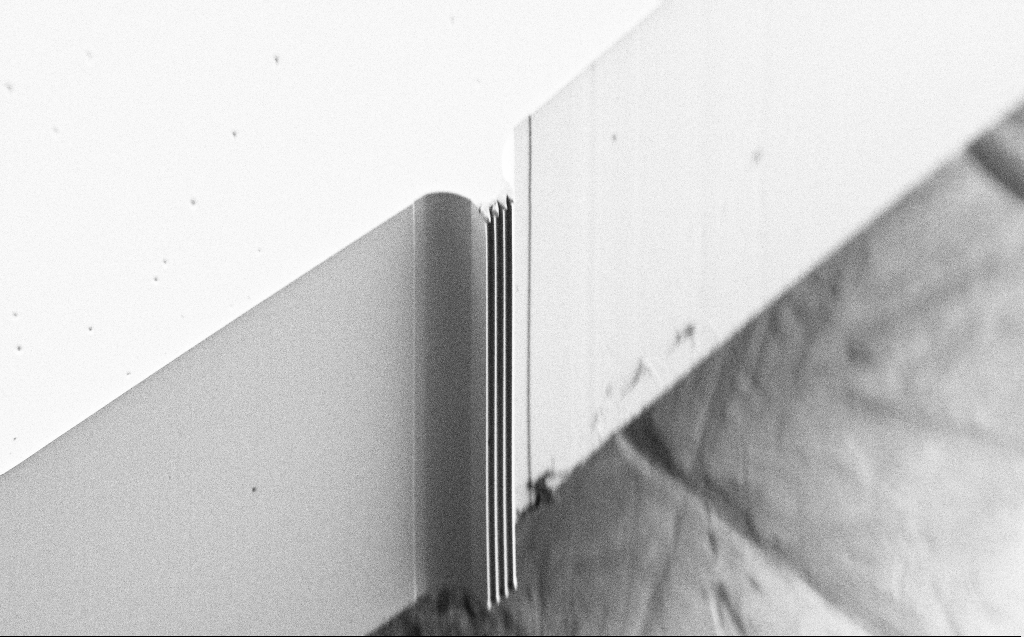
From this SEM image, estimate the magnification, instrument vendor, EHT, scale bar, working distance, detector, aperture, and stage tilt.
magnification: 0.345 K X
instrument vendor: Zeiss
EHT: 1 kV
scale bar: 100000 nm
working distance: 5 mm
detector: SE2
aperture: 30 µm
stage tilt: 44.6°